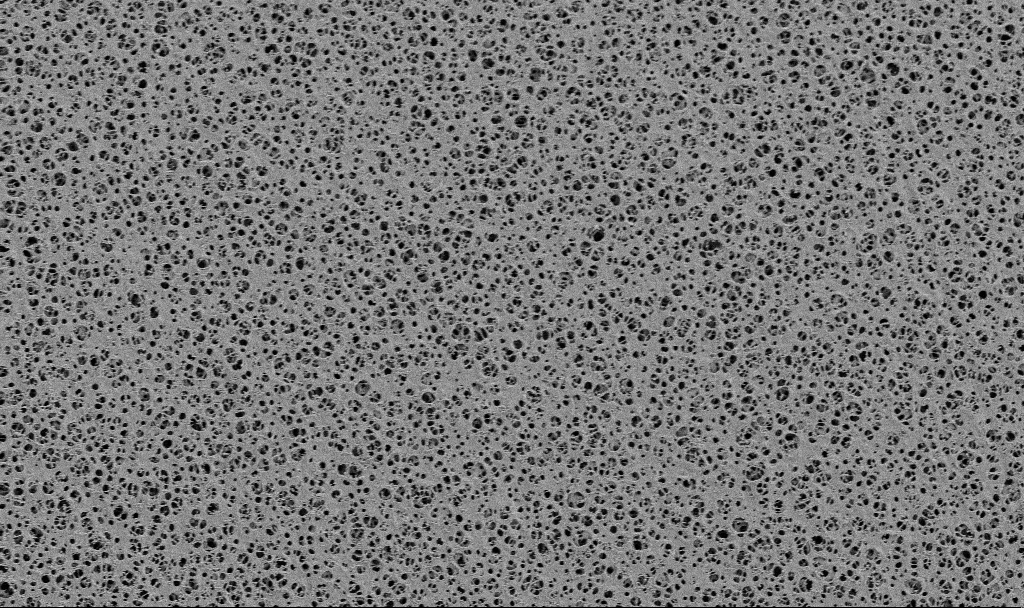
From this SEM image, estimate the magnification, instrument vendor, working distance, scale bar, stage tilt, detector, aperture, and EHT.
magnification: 2 K X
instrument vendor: Zeiss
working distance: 3.8 mm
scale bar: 10000 nm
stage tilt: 0°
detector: SE2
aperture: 30 µm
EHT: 2 kV